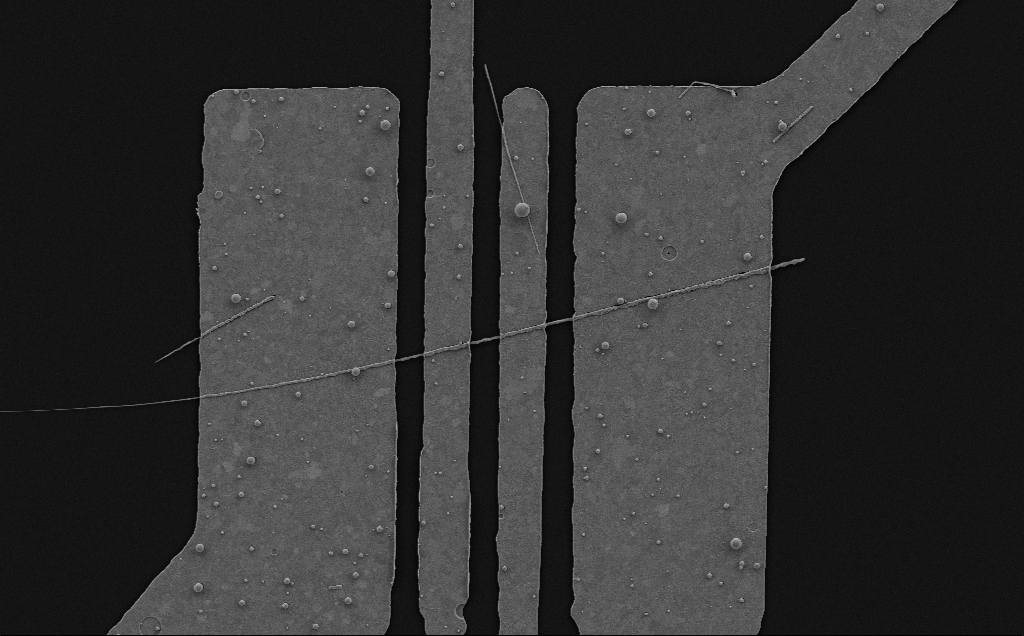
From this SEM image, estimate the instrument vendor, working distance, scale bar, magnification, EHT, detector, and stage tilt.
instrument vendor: Zeiss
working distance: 6 mm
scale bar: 10000 nm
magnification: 6.95 K X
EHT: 5 kV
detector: SE2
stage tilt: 0°